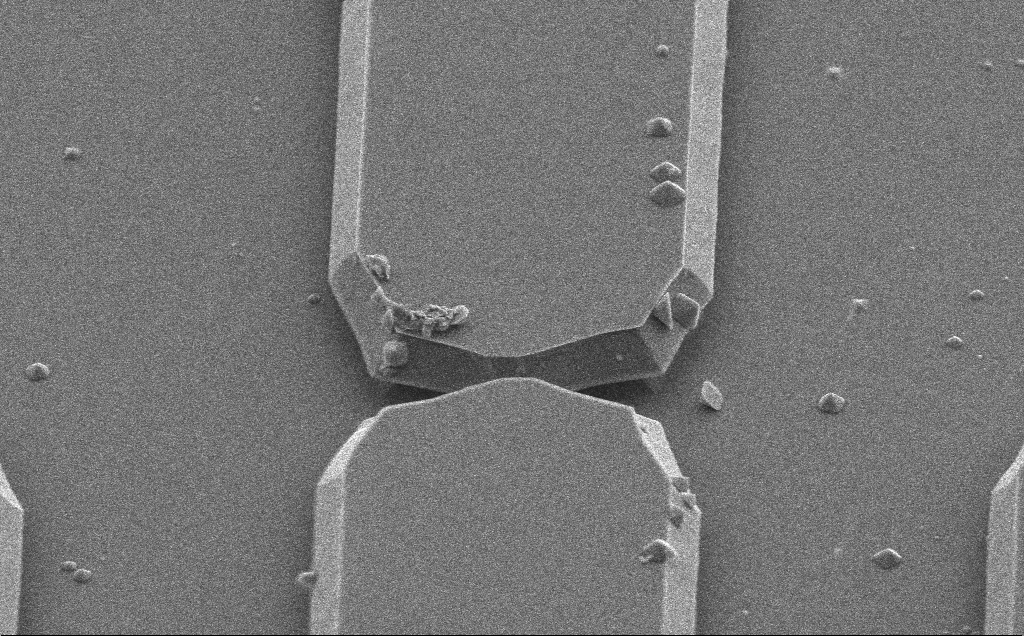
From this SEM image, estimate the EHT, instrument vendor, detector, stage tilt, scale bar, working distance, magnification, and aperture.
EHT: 5 kV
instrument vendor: Zeiss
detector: SE2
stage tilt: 50°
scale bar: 10000 nm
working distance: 10 mm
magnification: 6.21 K X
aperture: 30 µm